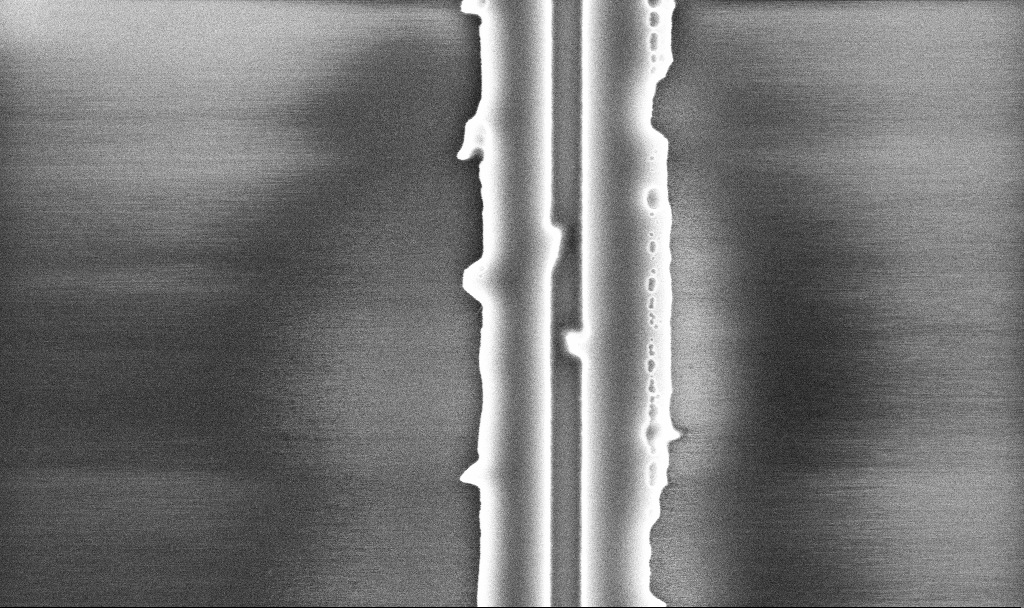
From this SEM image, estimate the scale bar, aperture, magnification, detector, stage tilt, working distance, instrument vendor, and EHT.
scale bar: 1000 nm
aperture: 30 µm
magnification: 51.06 K X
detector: InLens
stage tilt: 0°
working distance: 10.1 mm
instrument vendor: Zeiss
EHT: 5 kV